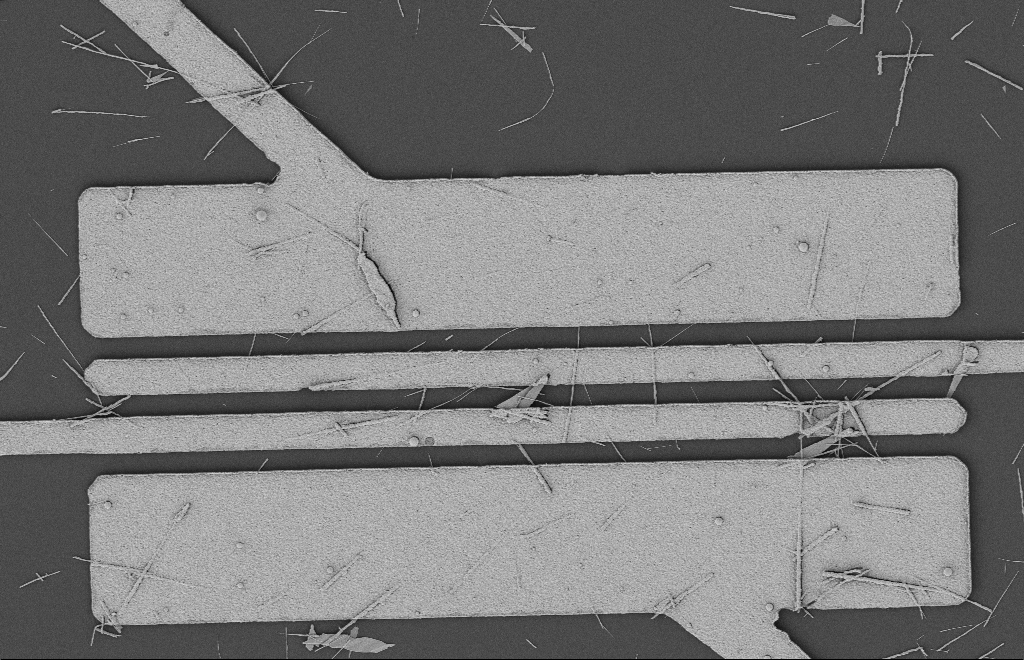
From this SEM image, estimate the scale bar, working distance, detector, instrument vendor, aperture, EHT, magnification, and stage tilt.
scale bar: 2000 nm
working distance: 12 mm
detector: SE2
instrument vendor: Zeiss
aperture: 20 µm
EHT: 2 kV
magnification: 5.26 K X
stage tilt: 0°